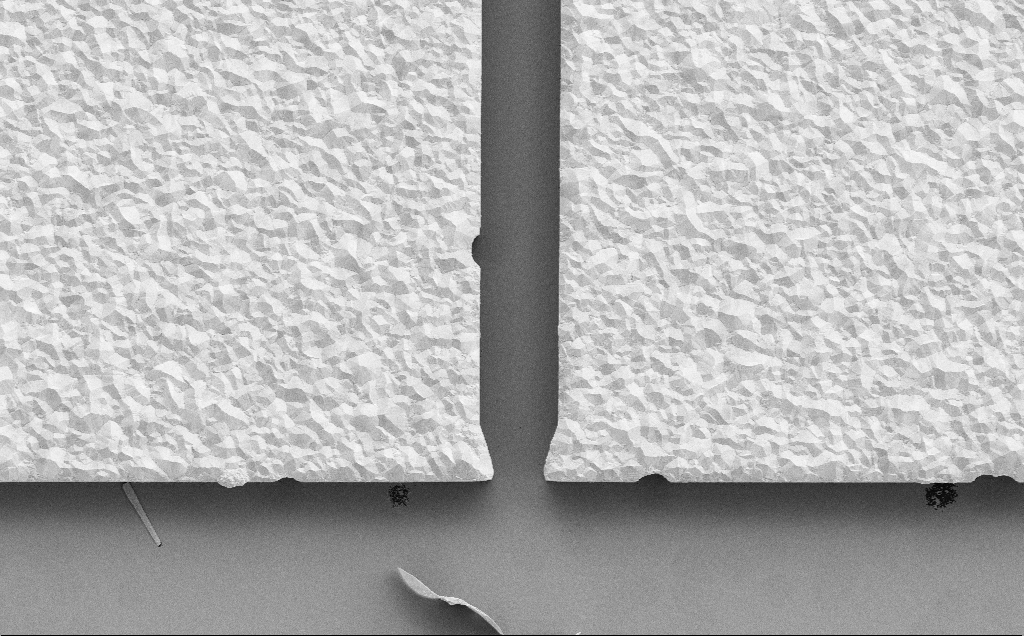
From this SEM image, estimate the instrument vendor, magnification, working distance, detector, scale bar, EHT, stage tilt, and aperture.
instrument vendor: Zeiss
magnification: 2.81 K X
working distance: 13 mm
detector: SE2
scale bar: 10000 nm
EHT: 5 kV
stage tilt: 0°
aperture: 30 µm